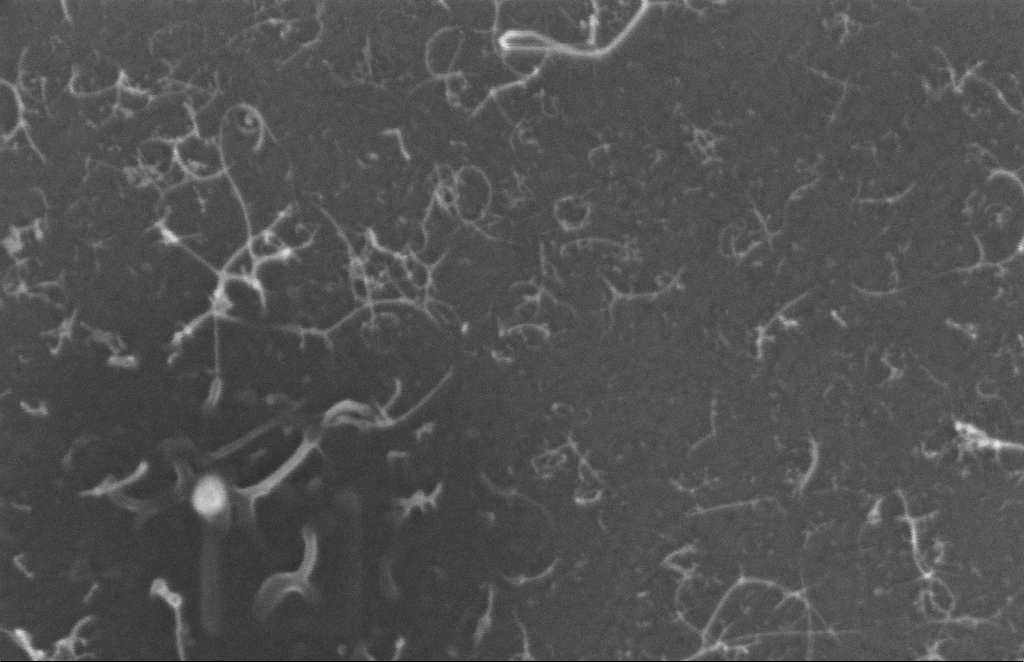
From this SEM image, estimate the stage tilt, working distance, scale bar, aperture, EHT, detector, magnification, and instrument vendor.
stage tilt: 0°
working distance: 8 mm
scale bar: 100 nm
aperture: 20 µm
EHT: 5 kV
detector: InLens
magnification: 268.53 K X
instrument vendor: Zeiss